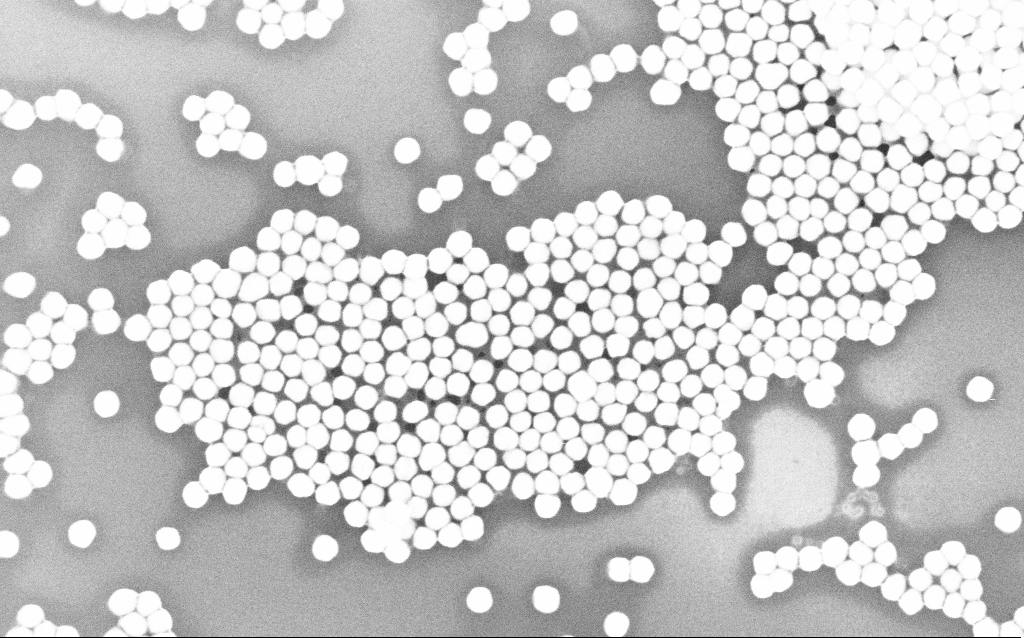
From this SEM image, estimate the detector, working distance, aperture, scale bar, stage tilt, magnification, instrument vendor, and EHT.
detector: InLens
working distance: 3.1 mm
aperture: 30 µm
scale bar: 200 nm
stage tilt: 0°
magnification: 142.26 K X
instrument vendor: Zeiss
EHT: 10 kV